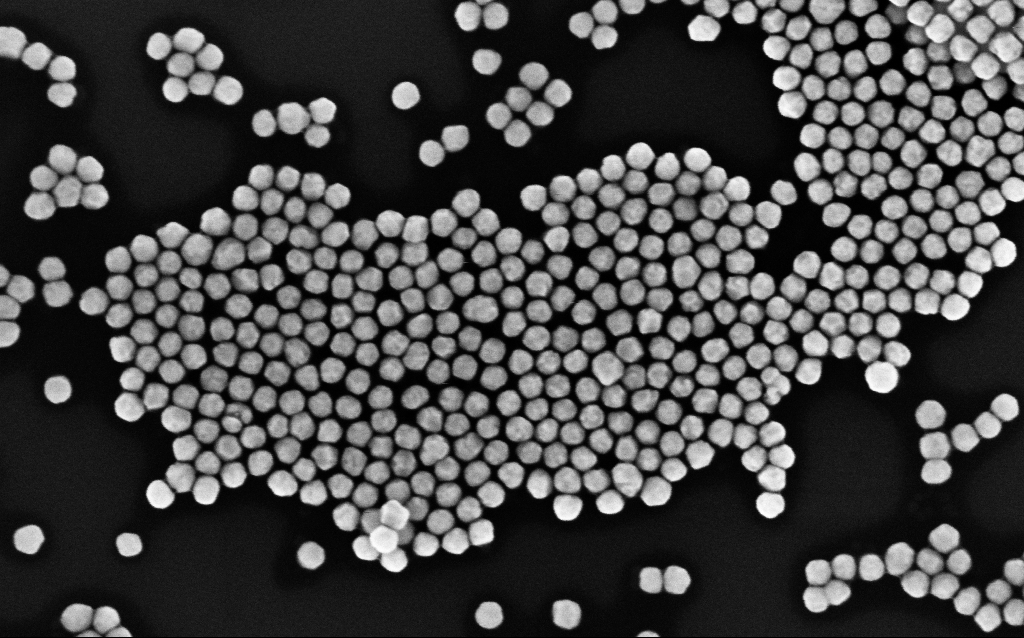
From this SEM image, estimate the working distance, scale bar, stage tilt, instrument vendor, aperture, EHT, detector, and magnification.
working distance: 3.1 mm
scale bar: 200 nm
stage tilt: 0°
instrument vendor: Zeiss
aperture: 30 µm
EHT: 8 kV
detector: InLens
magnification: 164.55 K X